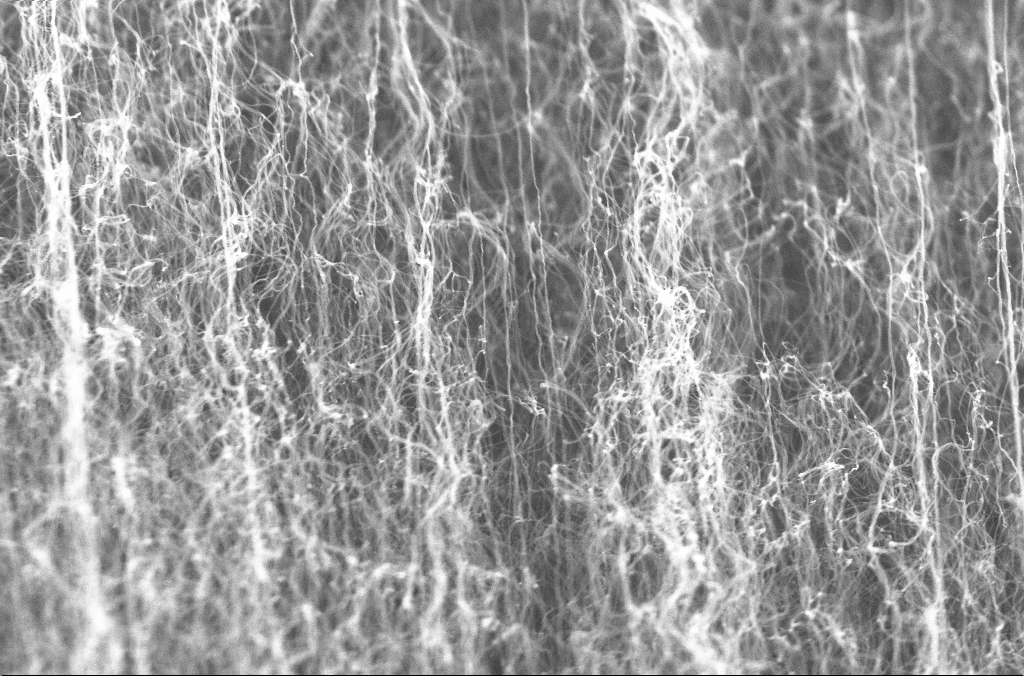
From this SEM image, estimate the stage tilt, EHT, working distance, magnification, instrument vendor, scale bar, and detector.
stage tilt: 0°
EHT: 20 kV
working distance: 4 mm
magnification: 50 K X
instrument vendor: Zeiss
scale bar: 1000 nm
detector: InLens